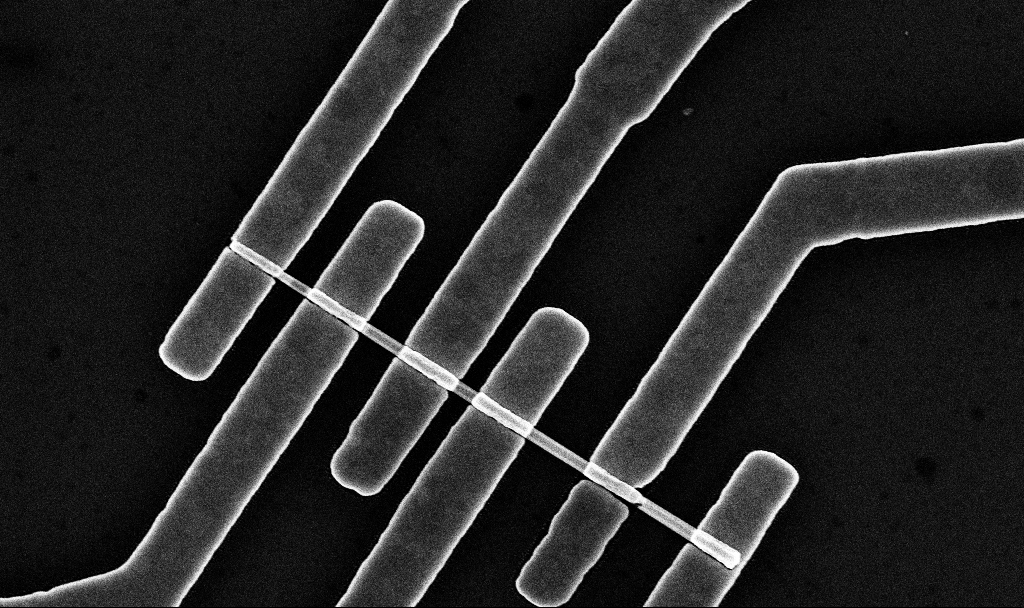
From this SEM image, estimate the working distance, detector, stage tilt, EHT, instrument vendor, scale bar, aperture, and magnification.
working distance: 7.7 mm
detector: InLens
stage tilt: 0°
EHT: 10 kV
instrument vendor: Zeiss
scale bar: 2000 nm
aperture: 30 µm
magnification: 35.26 K X